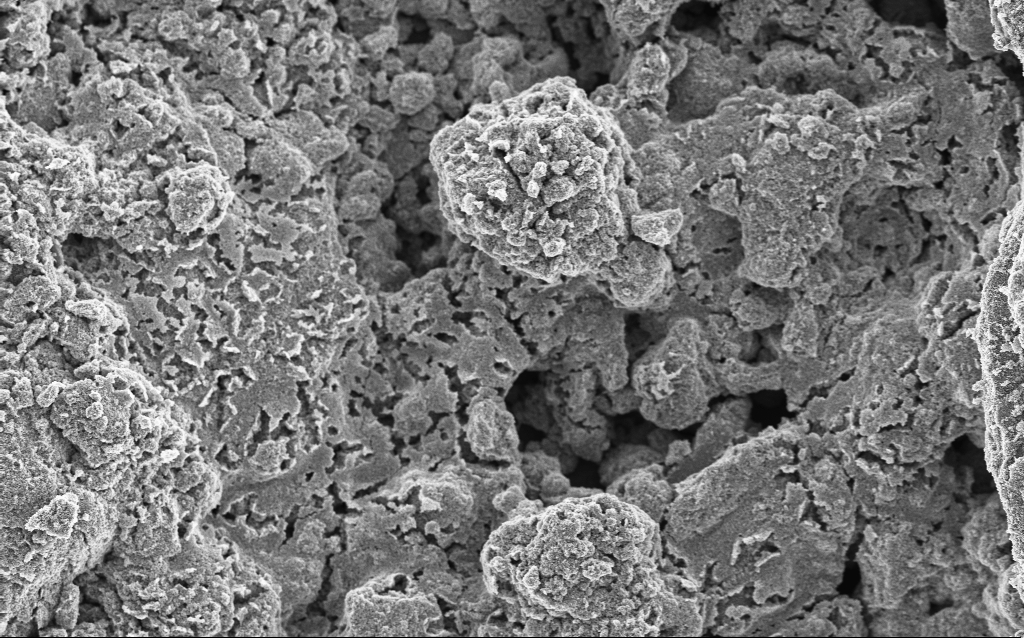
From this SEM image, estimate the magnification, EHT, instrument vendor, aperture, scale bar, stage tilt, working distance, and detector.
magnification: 2.6 K X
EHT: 5 kV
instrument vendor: Zeiss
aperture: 30 µm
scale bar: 10000 nm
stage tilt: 0°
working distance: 4.4 mm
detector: InLens